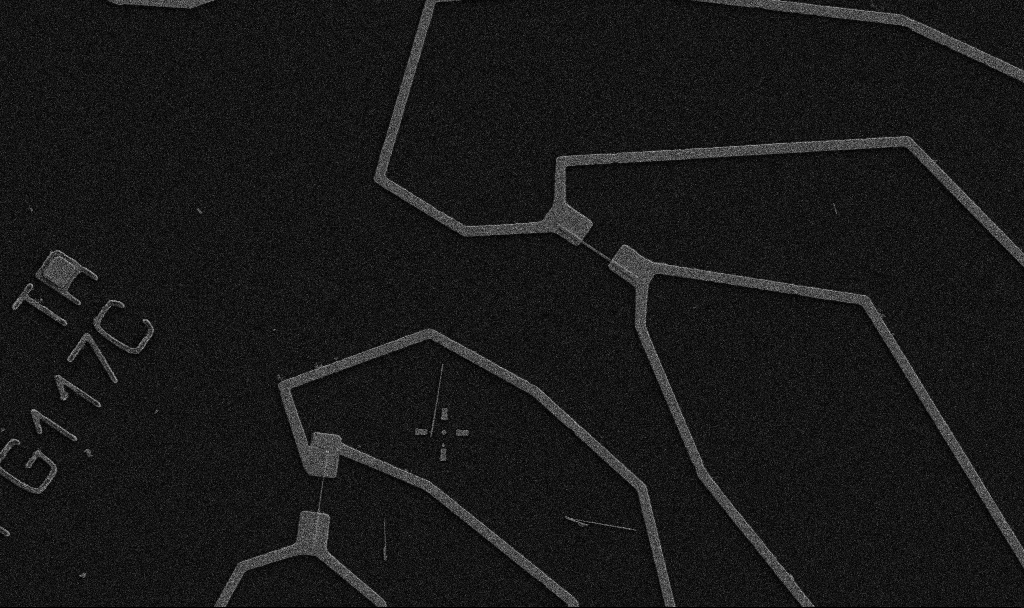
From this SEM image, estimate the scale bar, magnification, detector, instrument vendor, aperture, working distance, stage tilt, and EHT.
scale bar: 10000 nm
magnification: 5 K X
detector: SE2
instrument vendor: Zeiss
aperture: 30 µm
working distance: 10.7 mm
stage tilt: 0°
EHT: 5 kV